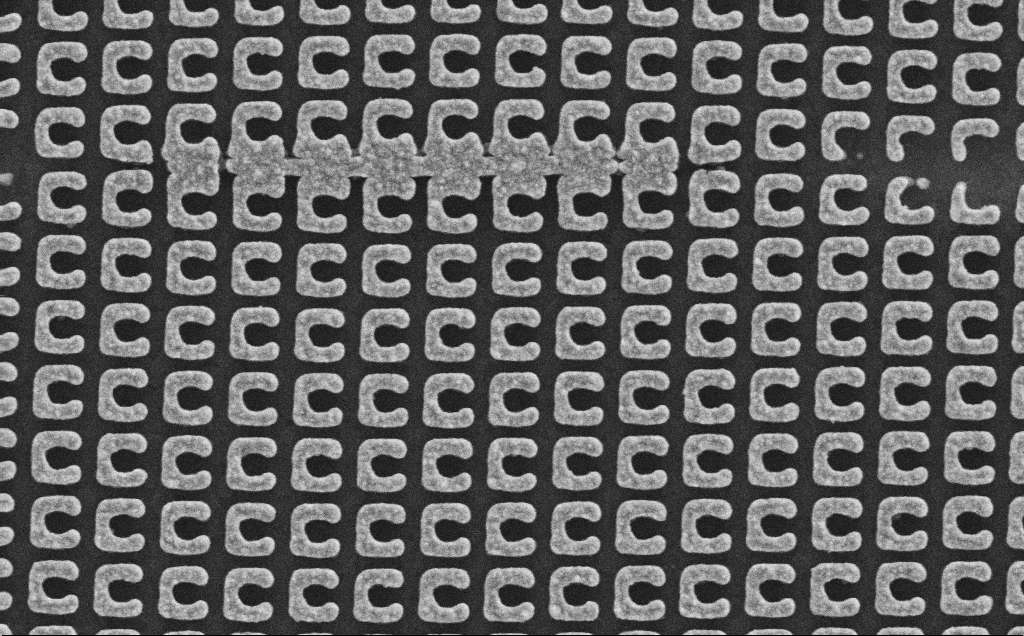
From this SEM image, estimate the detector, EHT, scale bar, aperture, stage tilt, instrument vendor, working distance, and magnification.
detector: SE2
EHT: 5 kV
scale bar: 1000 nm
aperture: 30 µm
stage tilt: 0°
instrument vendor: Zeiss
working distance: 2.3 mm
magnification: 52.01 K X